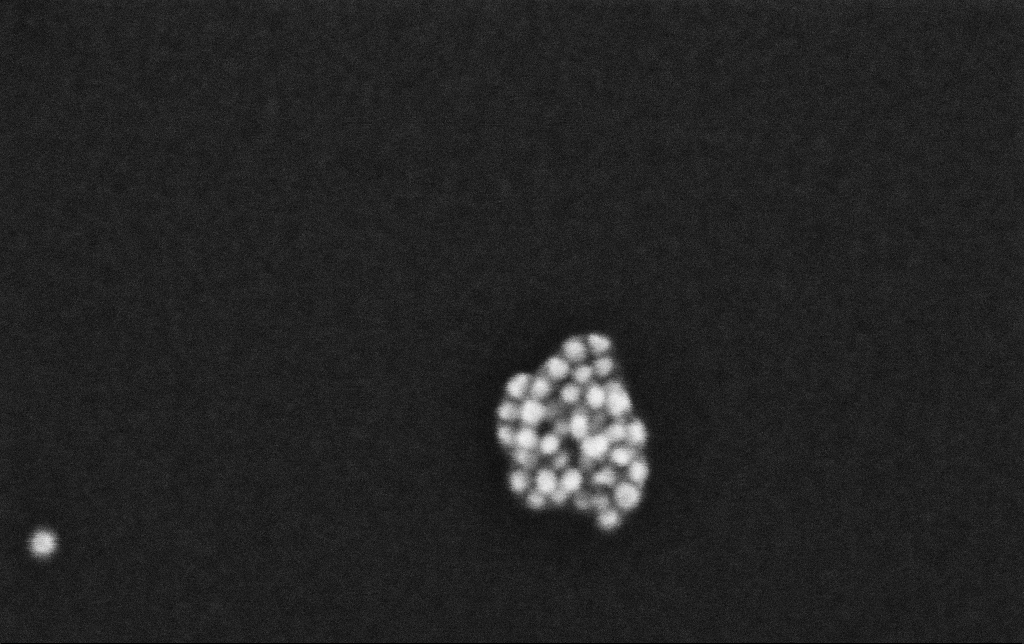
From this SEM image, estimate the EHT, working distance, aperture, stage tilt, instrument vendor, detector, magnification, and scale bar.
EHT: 10 kV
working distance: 3.3 mm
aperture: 30 µm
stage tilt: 0°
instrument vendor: Zeiss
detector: InLens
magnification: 623.33 K X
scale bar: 100 nm